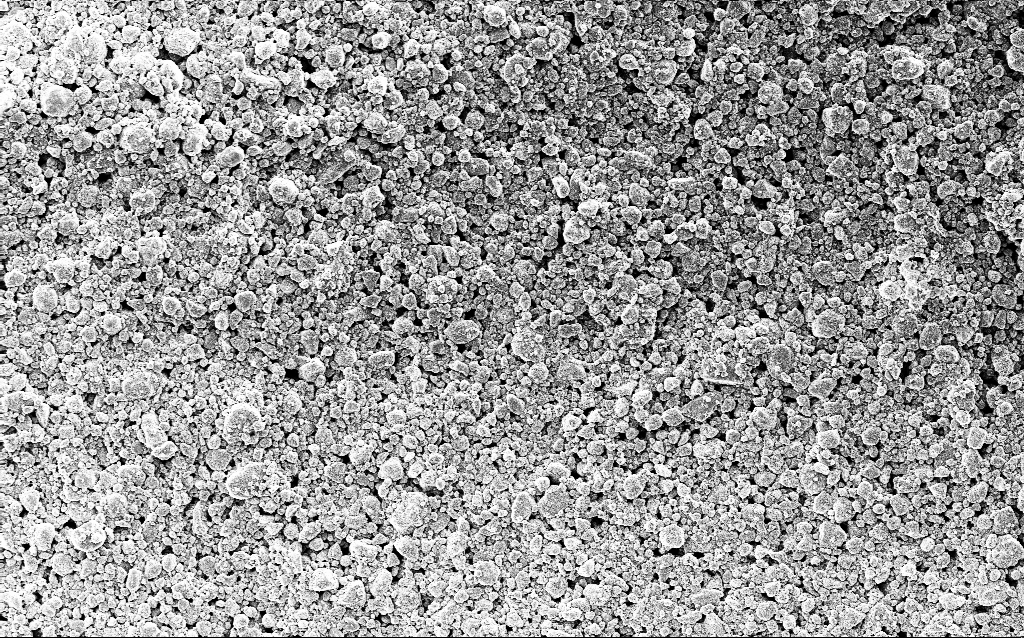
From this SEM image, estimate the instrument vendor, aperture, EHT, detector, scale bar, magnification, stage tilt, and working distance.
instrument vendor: Zeiss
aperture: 30 µm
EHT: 5 kV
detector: InLens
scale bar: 20000 nm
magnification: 1.42 K X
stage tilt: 0°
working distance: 1.6 mm